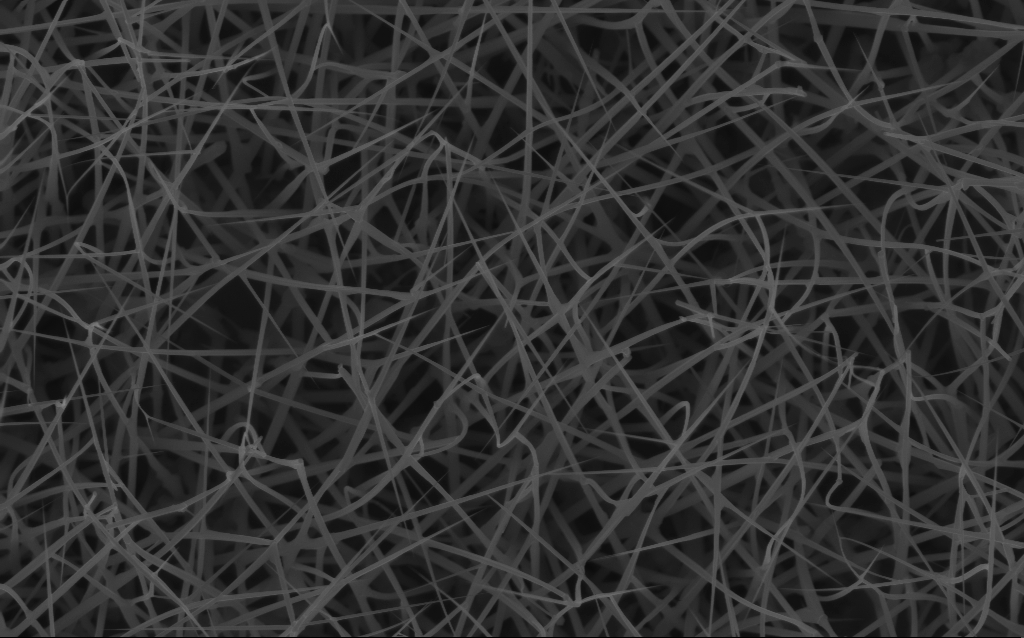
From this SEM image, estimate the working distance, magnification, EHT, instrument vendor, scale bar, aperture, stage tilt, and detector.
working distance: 6 mm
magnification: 20 K X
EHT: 10 kV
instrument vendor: Zeiss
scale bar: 2000 nm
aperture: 30 µm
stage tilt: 0°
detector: InLens